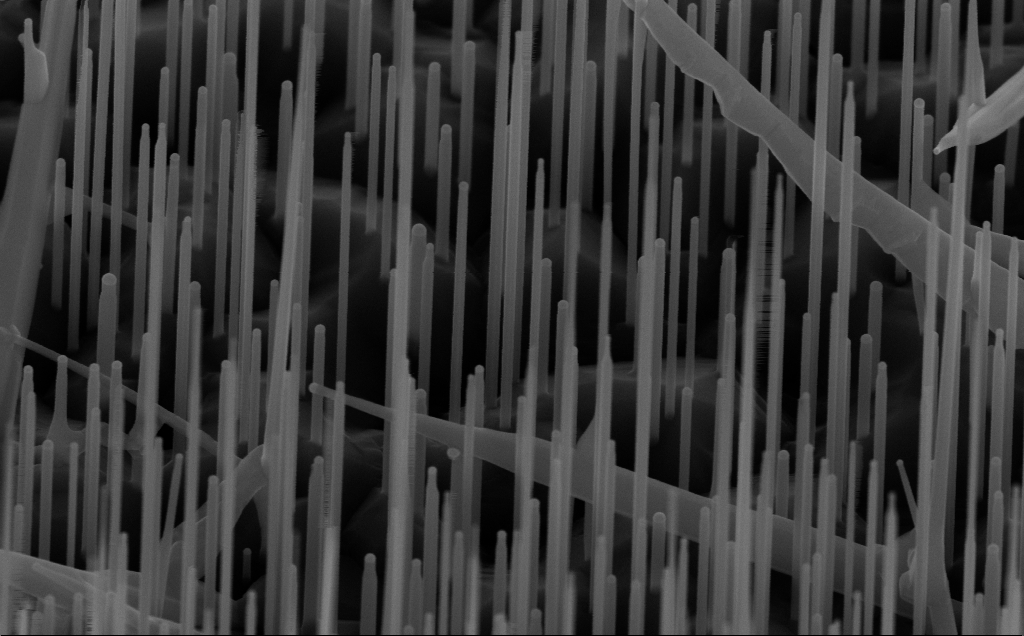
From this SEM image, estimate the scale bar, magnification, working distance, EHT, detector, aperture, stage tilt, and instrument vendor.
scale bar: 200 nm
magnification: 80 K X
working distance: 7 mm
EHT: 10 kV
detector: InLens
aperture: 30 µm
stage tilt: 45°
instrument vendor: Zeiss